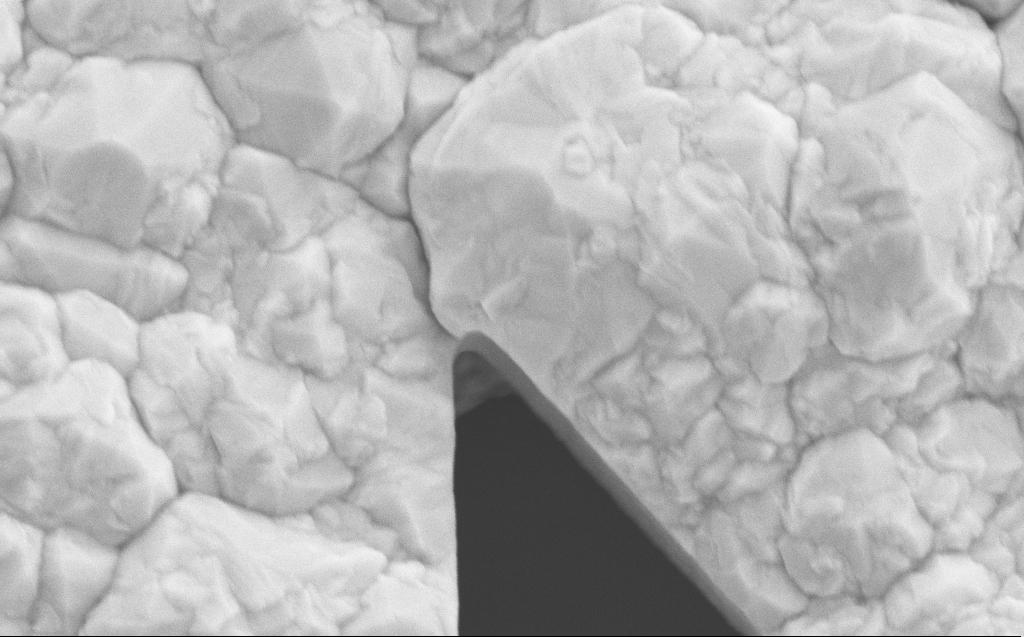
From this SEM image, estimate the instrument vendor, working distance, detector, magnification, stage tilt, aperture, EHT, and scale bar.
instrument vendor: Zeiss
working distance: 9 mm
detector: SE2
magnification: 59.76 K X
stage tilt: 0°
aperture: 30 µm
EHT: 10 kV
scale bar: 1000 nm